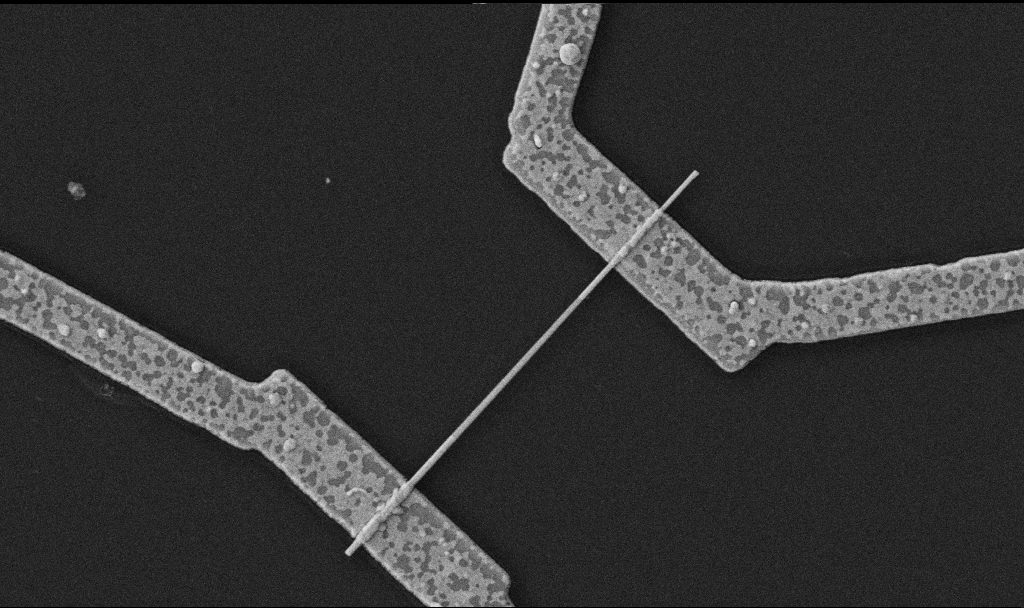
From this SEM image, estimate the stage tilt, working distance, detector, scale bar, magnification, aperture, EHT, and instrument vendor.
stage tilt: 0°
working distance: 10.7 mm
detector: SE2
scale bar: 1000 nm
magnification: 30 K X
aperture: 30 µm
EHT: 5 kV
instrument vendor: Zeiss